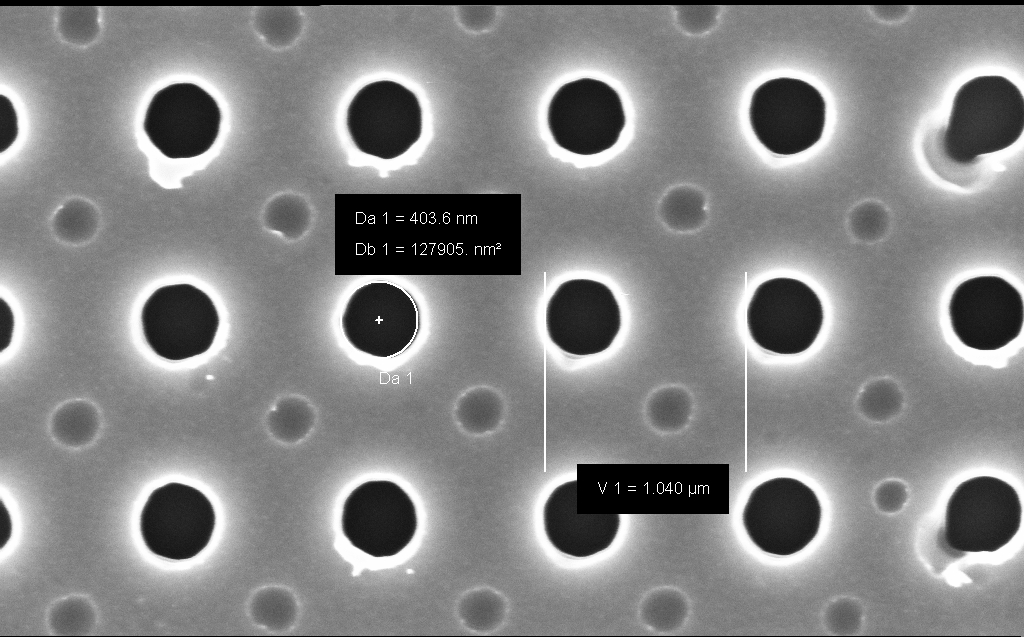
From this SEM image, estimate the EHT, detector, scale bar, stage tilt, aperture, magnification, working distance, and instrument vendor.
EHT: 5 kV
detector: InLens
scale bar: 1000 nm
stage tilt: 0°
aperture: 30 µm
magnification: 70.97 K X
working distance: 4 mm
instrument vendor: Zeiss